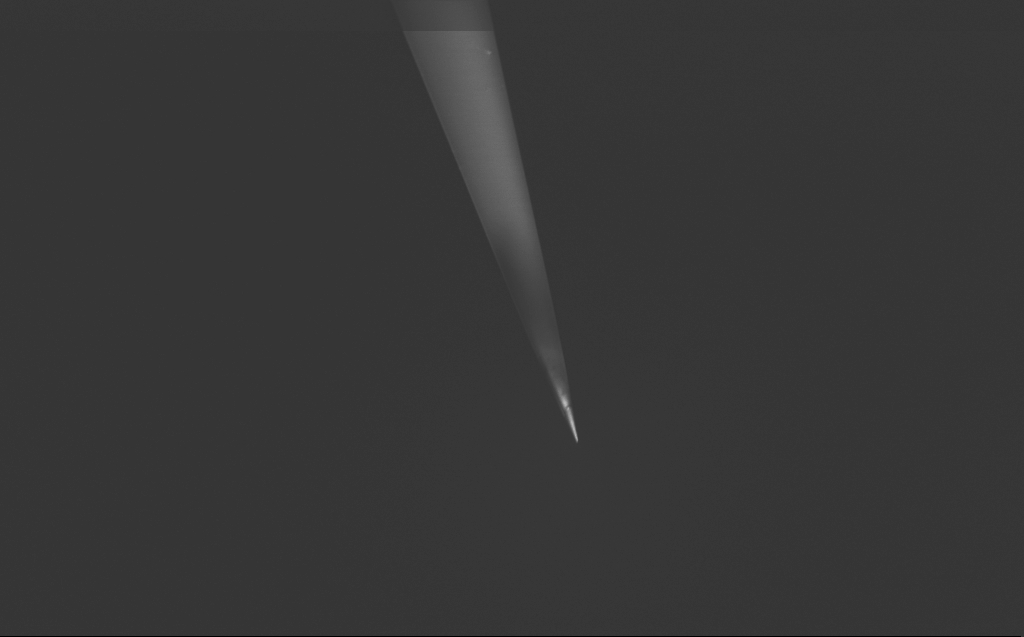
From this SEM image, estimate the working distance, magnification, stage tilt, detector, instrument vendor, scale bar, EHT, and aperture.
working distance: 5 mm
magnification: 5 K X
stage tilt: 39.3°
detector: InLens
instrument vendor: Zeiss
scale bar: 10000 nm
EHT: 0.8 kV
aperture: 30 µm